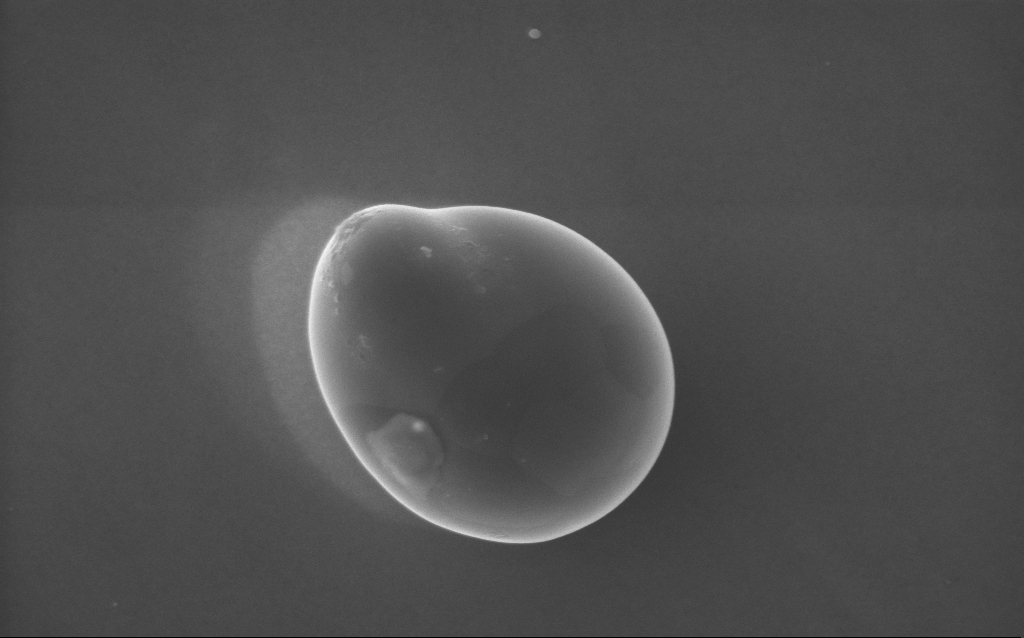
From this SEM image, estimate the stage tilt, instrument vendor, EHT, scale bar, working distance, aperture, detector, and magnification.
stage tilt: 0°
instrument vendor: Zeiss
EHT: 5 kV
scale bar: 200 nm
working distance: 3 mm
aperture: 30 µm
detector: InLens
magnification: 80 K X